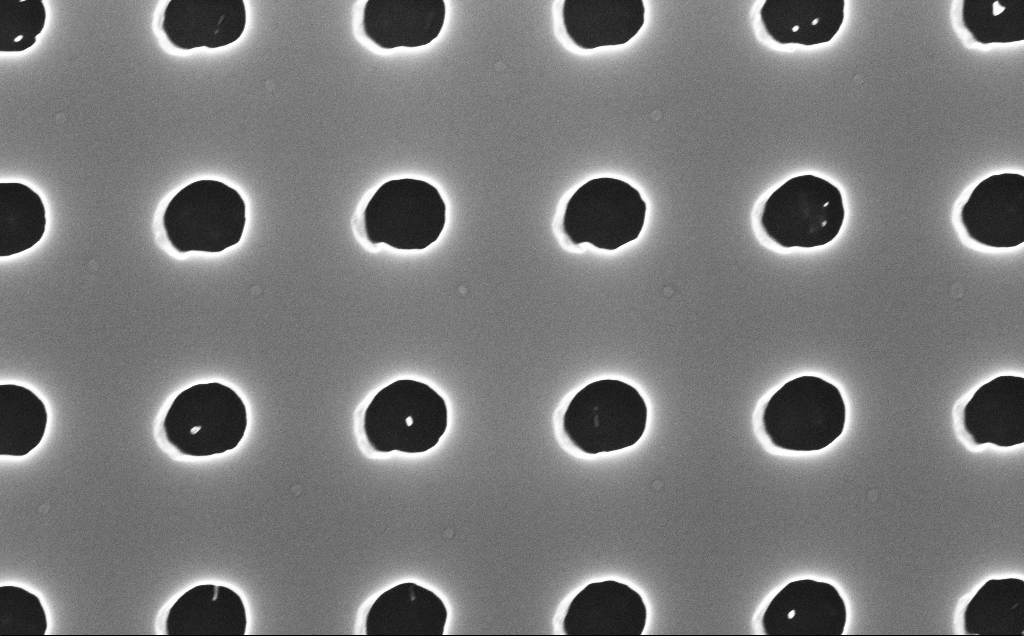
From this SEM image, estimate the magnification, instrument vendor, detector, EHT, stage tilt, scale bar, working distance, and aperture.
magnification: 70 K X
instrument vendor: Zeiss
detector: InLens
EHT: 10 kV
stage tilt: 0°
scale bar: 1000 nm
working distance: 4 mm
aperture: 30 µm